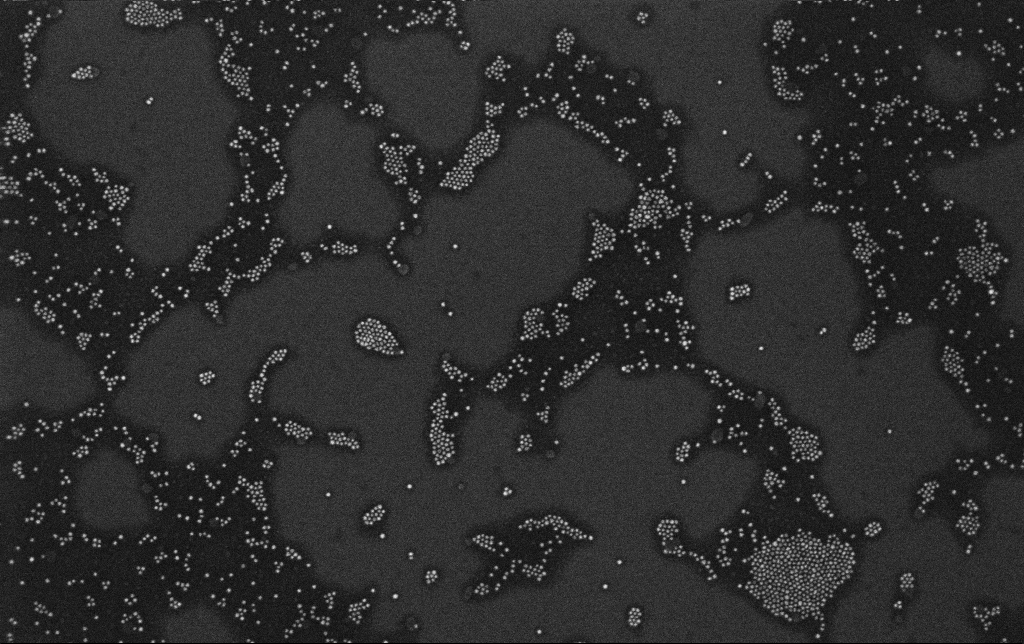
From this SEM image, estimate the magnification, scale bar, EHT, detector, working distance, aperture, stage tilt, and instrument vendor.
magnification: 100 K X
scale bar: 200 nm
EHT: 10 kV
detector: InLens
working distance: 3.4 mm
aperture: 30 µm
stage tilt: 0°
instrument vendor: Zeiss